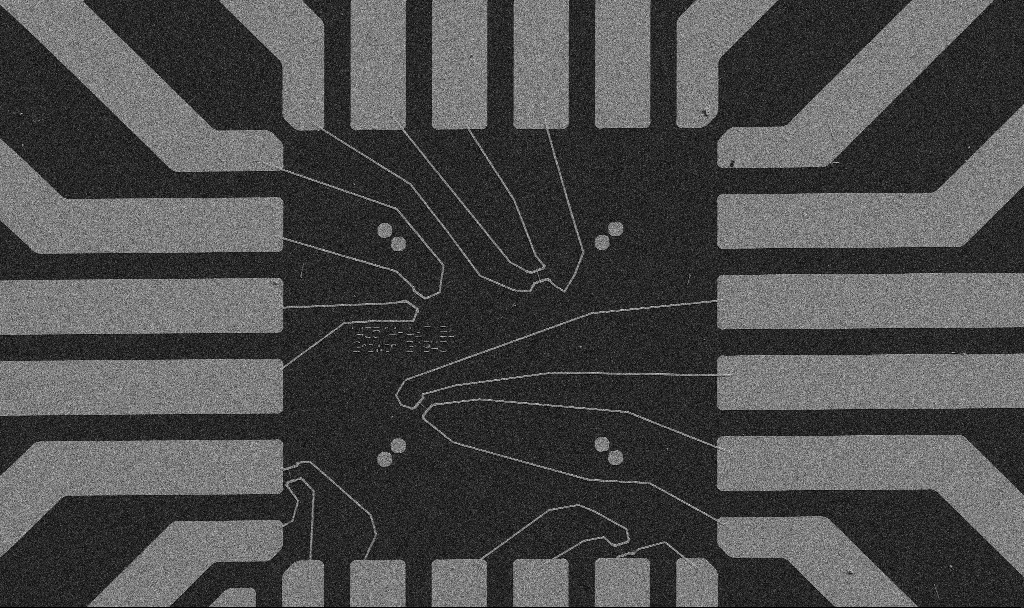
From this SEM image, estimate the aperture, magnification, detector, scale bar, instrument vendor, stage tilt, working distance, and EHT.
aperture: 30 µm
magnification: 1 K X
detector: SE2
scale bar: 20000 nm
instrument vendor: Zeiss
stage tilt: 0°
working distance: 10.7 mm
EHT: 5 kV